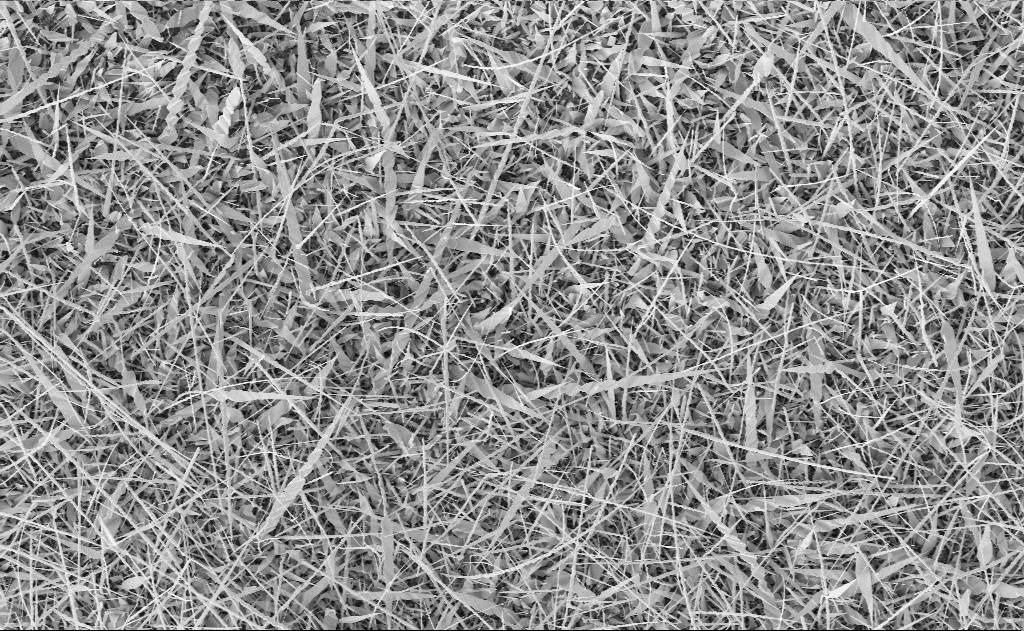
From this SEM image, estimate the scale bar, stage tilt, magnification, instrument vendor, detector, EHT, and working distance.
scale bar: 10000 nm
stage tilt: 0°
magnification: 5 K X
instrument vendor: Zeiss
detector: InLens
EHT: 10 kV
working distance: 19 mm